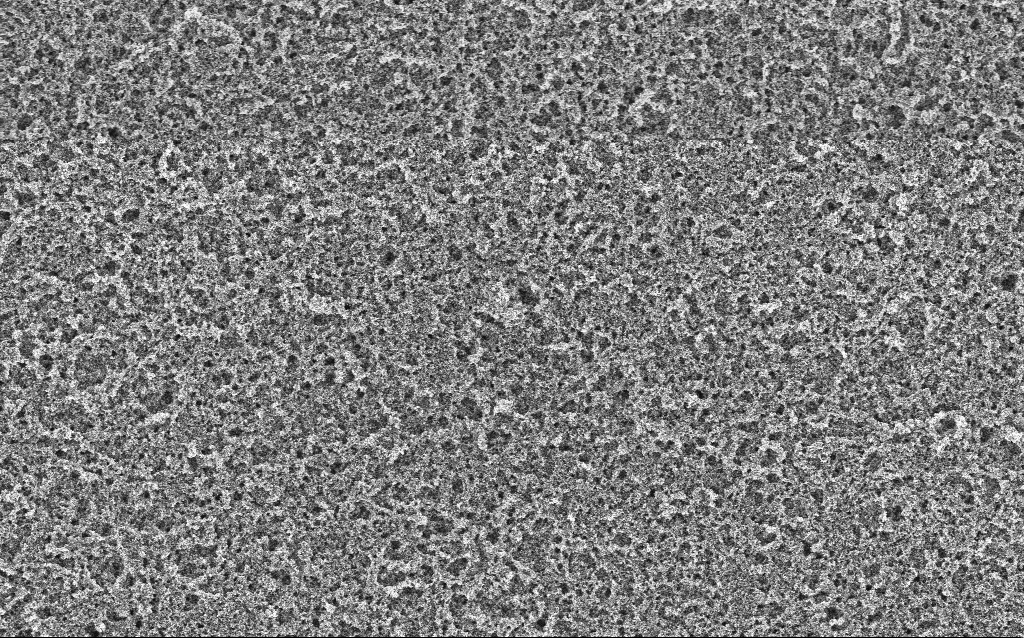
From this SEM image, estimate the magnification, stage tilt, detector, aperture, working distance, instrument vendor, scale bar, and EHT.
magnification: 6.61 K X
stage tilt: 0°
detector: InLens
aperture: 30 µm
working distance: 4.4 mm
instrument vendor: Zeiss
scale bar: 10000 nm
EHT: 5 kV